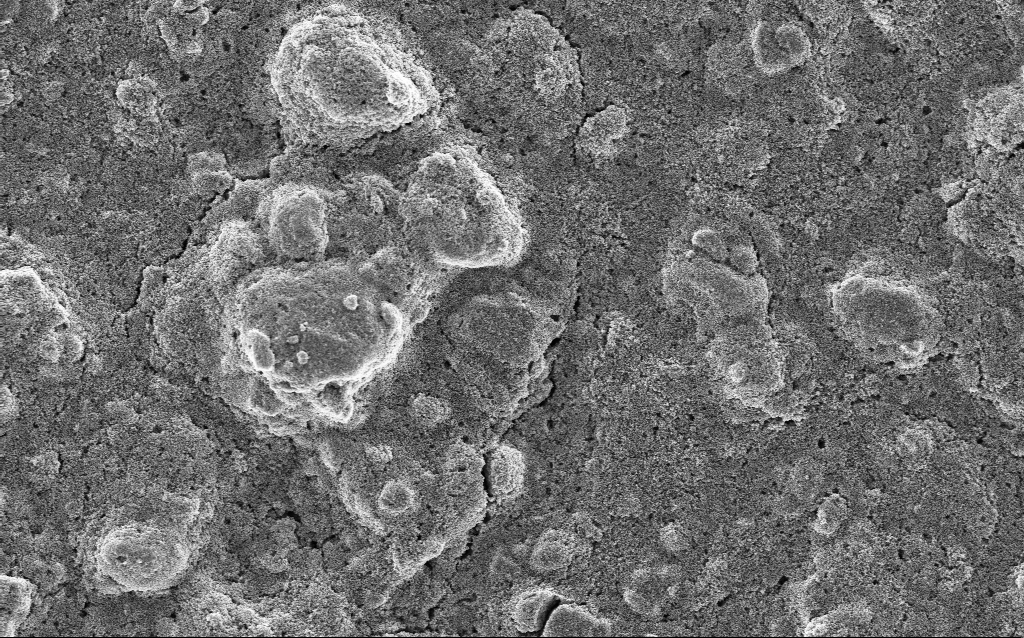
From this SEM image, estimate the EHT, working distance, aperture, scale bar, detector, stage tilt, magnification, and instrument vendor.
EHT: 5 kV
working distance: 3 mm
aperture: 30 µm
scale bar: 10000 nm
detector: InLens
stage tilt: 0°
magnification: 5 K X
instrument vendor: Zeiss